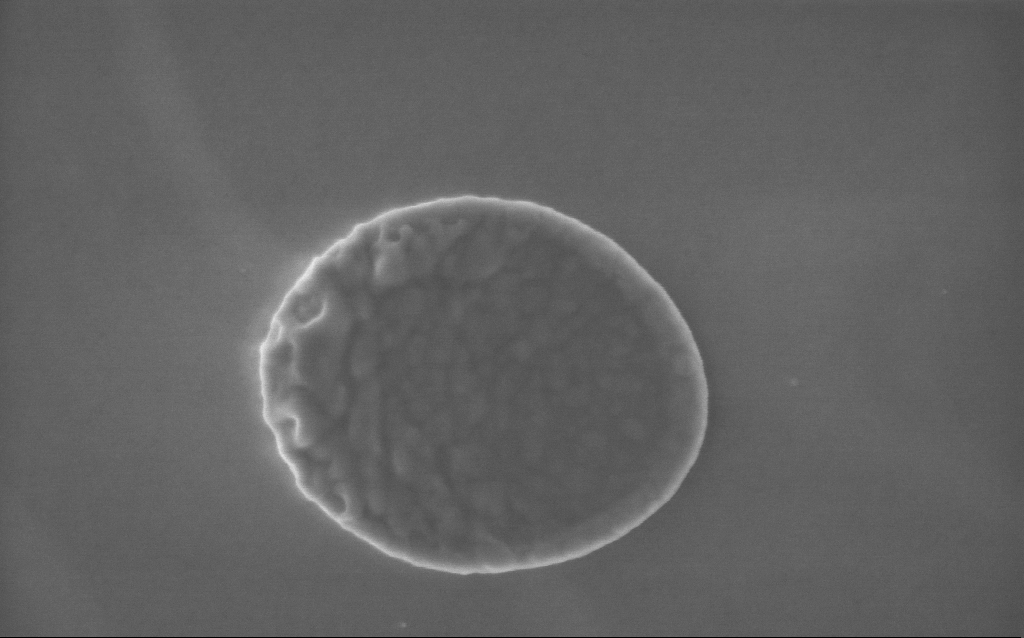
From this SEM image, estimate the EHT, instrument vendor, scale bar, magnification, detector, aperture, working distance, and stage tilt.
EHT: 5 kV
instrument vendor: Zeiss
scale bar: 200 nm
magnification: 91 K X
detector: InLens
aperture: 30 µm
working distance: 3 mm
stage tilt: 0°